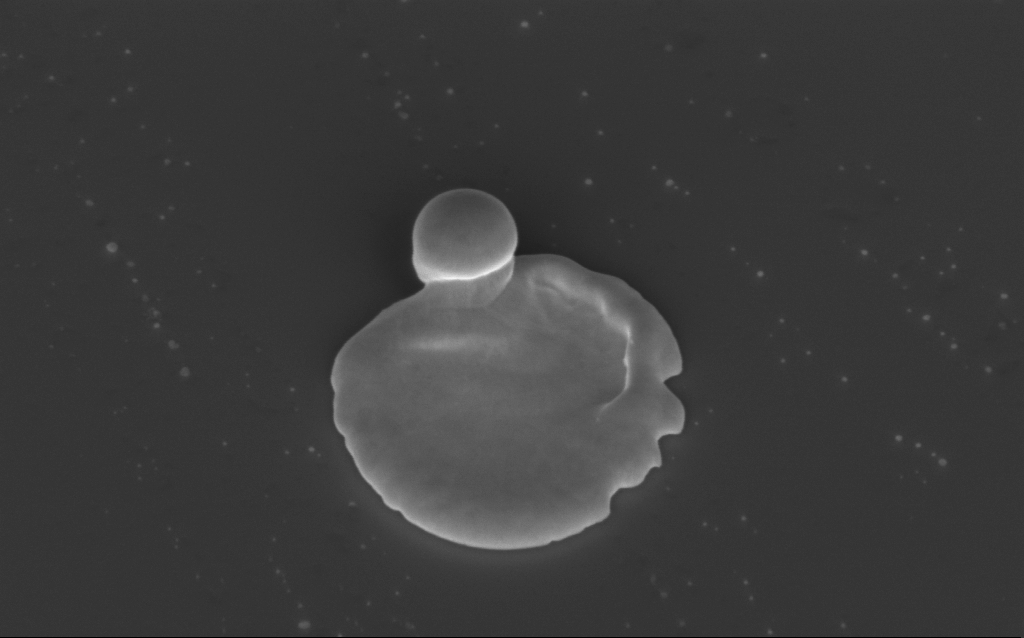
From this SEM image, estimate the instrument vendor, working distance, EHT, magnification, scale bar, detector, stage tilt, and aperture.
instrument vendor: Zeiss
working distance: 5 mm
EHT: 3 kV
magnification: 45.95 K X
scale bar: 1000 nm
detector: InLens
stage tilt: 40°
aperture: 30 µm